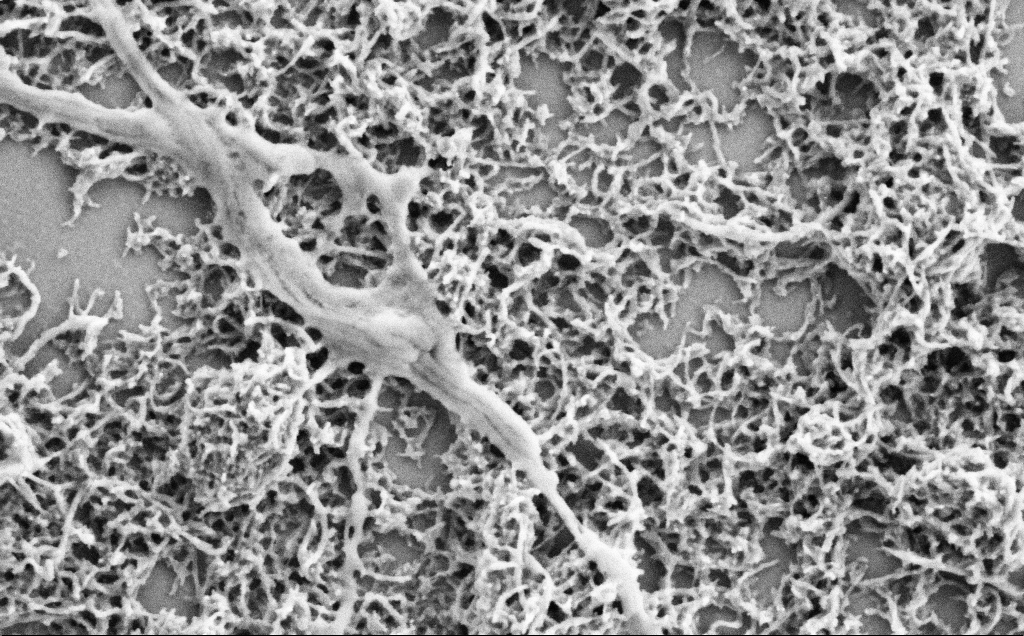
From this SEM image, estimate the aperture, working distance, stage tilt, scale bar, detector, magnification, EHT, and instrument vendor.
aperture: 30 µm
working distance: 7.1 mm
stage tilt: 0°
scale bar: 1000 nm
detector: SE2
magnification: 50 K X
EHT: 2 kV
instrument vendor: Zeiss